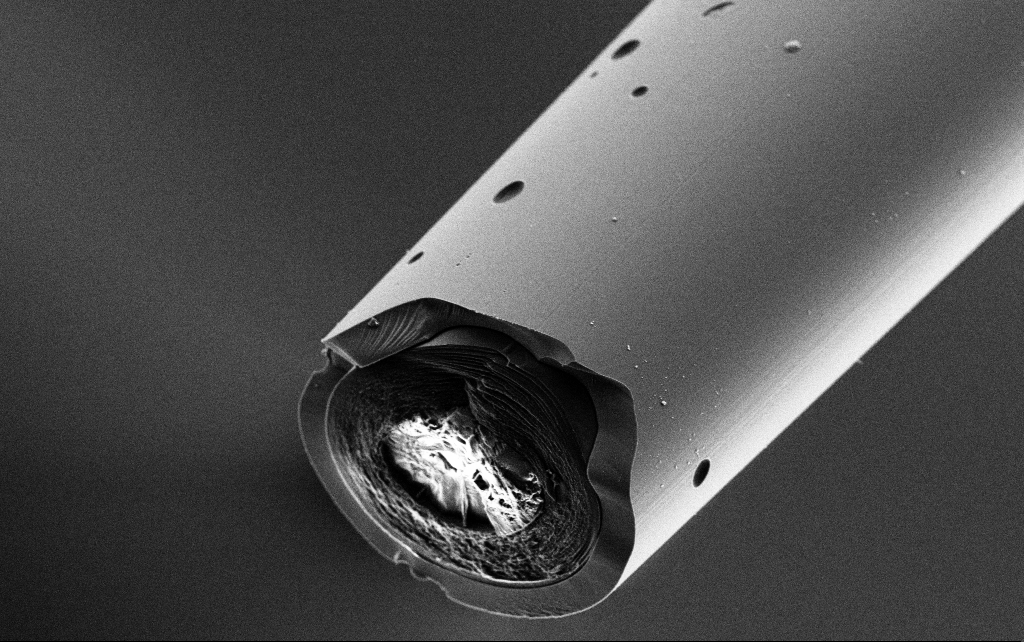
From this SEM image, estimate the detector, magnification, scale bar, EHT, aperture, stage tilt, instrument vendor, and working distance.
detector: SE2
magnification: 5 K X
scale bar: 10000 nm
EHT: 3 kV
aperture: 30 µm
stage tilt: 45°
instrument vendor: Zeiss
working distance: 7.8 mm